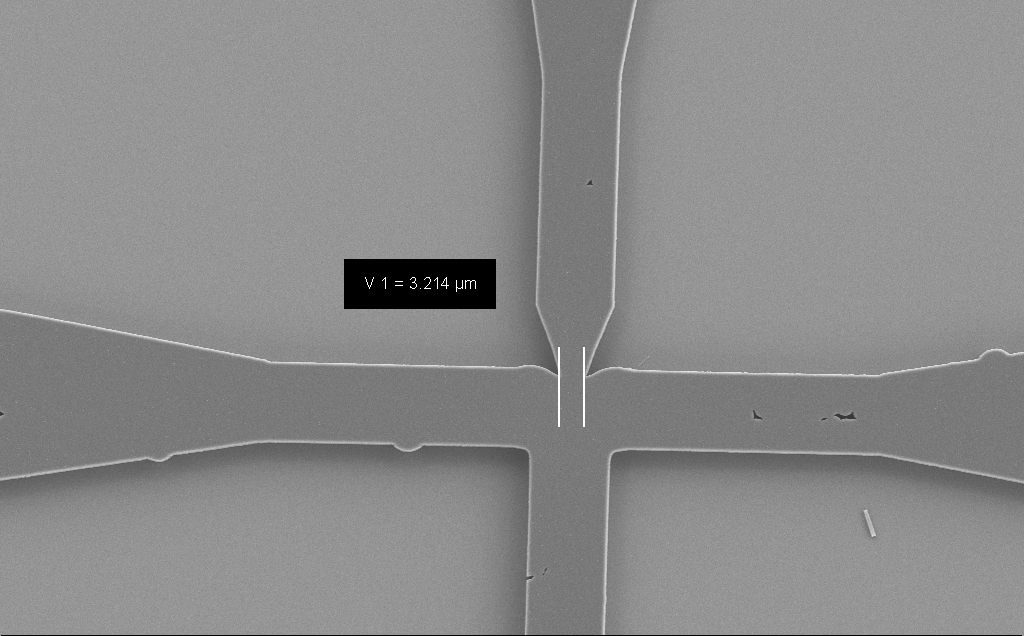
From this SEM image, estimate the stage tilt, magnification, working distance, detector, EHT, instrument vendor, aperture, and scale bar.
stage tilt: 0°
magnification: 2.86 K X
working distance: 9 mm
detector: SE2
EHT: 5 kV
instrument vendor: Zeiss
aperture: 30 µm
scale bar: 10000 nm